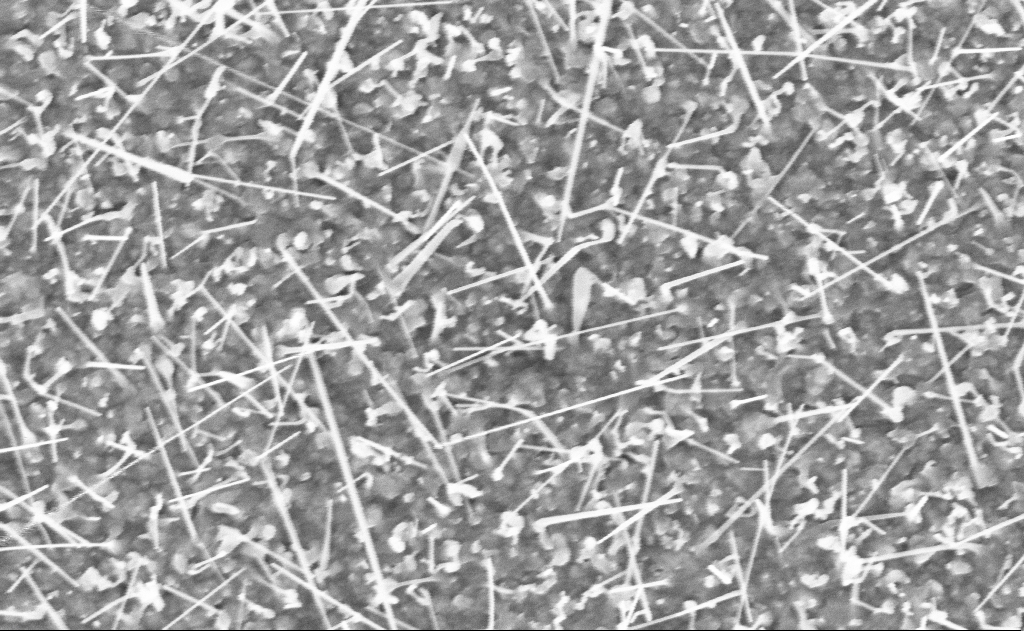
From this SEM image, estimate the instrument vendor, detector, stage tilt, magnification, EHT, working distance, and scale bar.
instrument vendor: Zeiss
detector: InLens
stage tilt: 0°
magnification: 40 K X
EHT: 10 kV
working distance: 20 mm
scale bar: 1000 nm